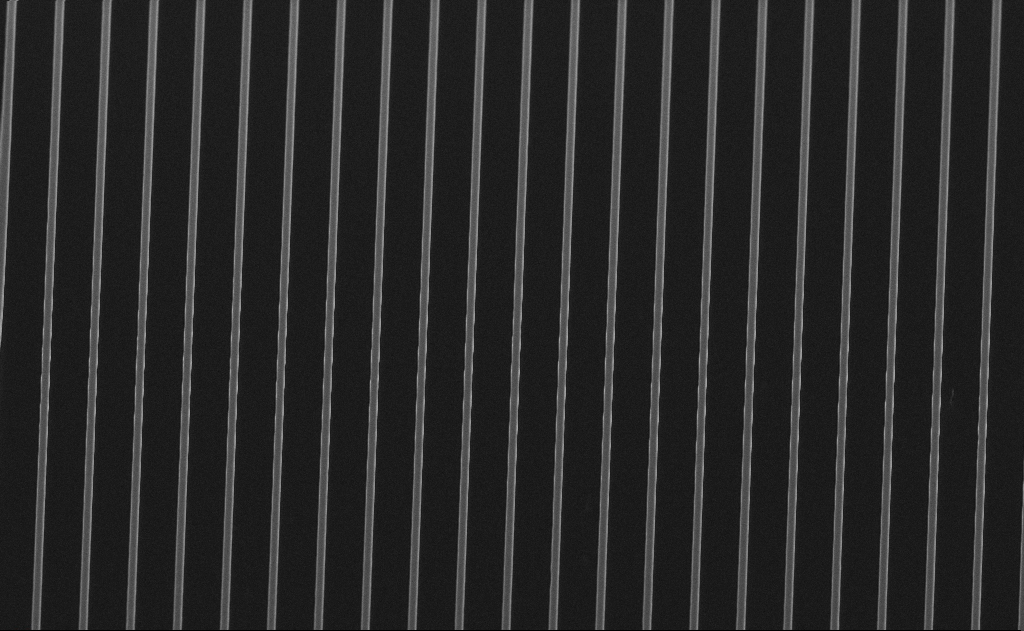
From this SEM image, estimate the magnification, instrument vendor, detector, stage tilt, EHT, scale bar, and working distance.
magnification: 2.16 K X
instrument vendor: Zeiss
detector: SE2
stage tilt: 50°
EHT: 6 kV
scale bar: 20000 nm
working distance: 12 mm